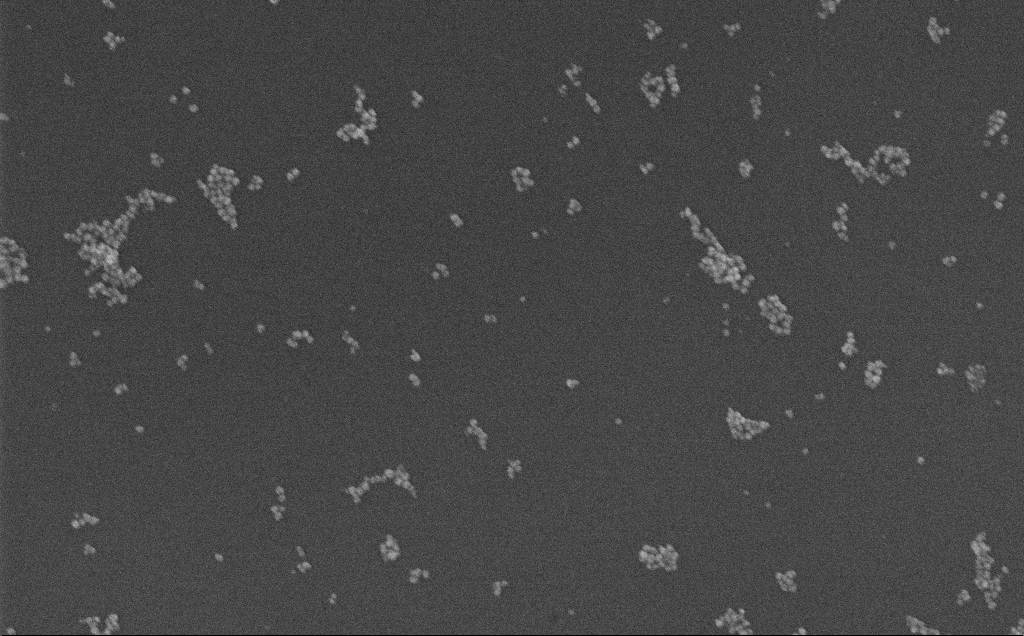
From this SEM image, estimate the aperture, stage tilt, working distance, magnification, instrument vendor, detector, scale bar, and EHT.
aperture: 30 µm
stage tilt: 0°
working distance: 3.7 mm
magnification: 104.25 K X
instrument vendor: Zeiss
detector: InLens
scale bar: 200 nm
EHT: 10 kV